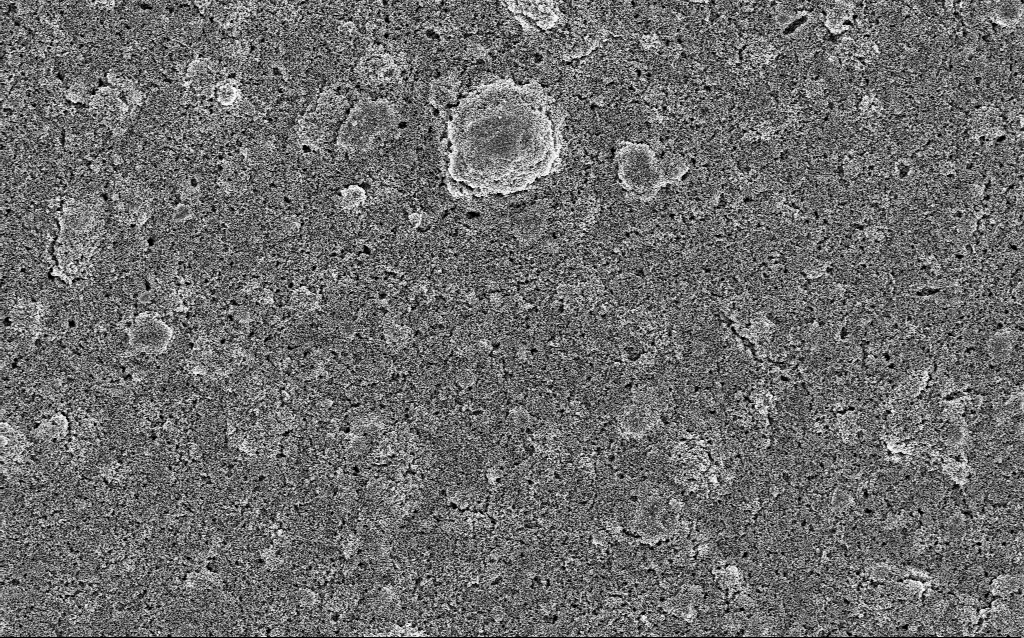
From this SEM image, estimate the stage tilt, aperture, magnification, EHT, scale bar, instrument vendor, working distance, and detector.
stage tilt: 0°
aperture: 30 µm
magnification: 5 K X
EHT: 5 kV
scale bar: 10000 nm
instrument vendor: Zeiss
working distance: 5.3 mm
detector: InLens